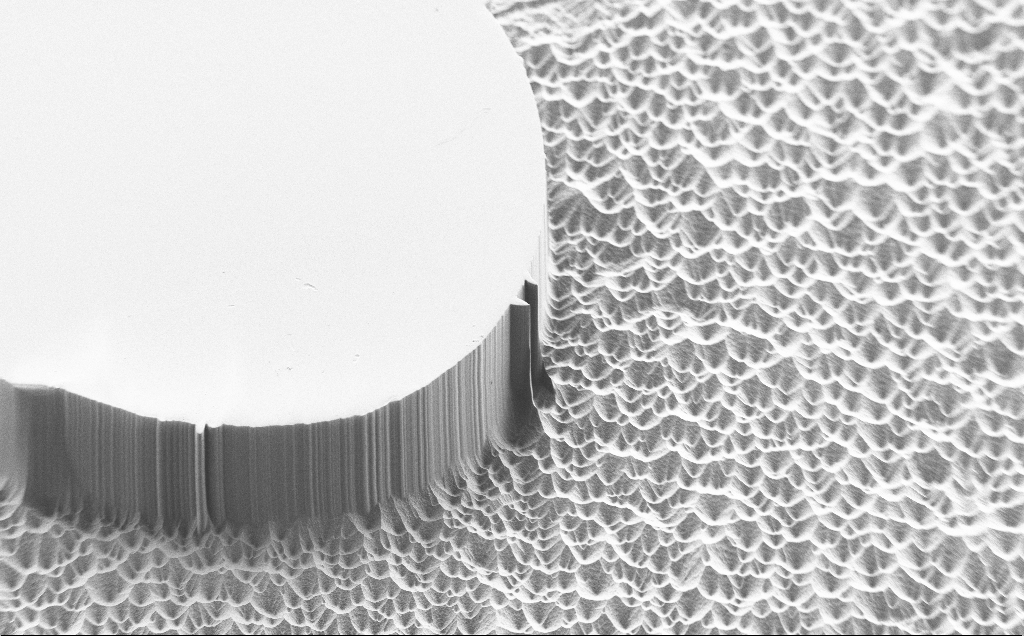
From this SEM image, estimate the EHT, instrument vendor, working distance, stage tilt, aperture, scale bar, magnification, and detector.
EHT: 1.2 kV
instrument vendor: Zeiss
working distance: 6 mm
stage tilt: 45°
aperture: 30 µm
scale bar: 100000 nm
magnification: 0.483 K X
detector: SE2